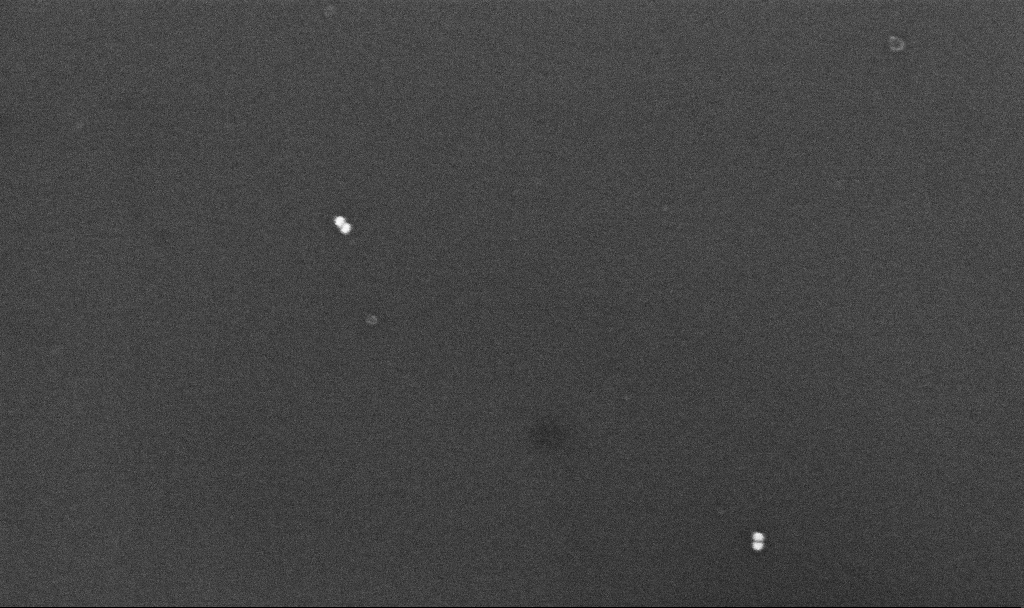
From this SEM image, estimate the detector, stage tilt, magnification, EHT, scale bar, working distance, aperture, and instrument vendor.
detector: InLens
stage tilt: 0°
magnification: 212.13 K X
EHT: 10 kV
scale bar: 200 nm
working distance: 4.3 mm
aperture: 30 µm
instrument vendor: Zeiss